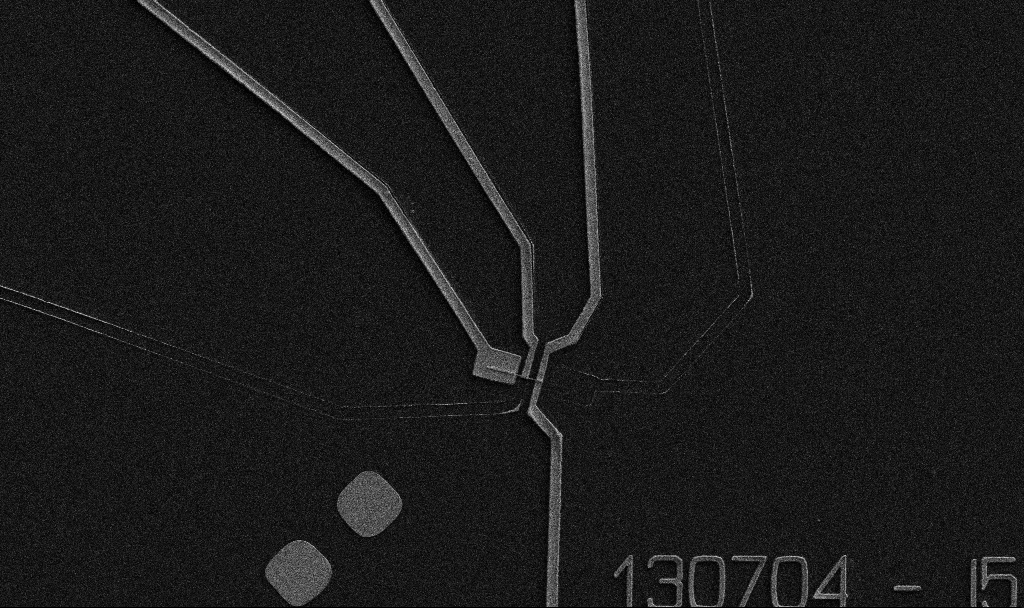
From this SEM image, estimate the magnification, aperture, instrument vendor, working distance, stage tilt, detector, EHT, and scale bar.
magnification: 5 K X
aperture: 30 µm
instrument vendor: Zeiss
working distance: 10.7 mm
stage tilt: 0°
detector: SE2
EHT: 5 kV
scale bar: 10000 nm